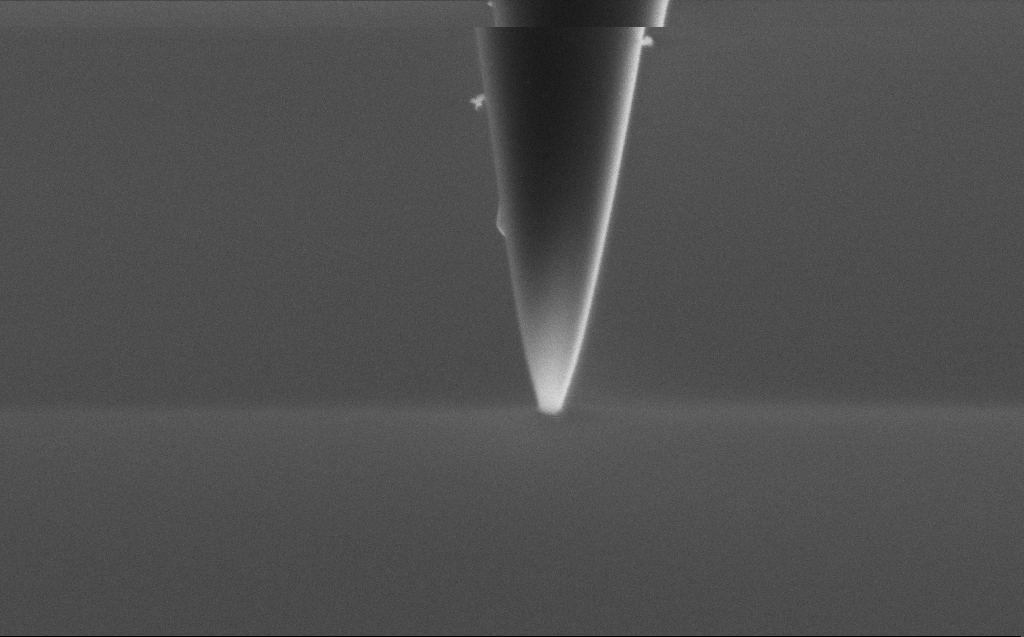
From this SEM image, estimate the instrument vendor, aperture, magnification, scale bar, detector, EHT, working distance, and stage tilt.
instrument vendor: Zeiss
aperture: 30 µm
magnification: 90.26 K X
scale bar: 200 nm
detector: SE2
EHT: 2 kV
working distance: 3 mm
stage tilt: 45.1°